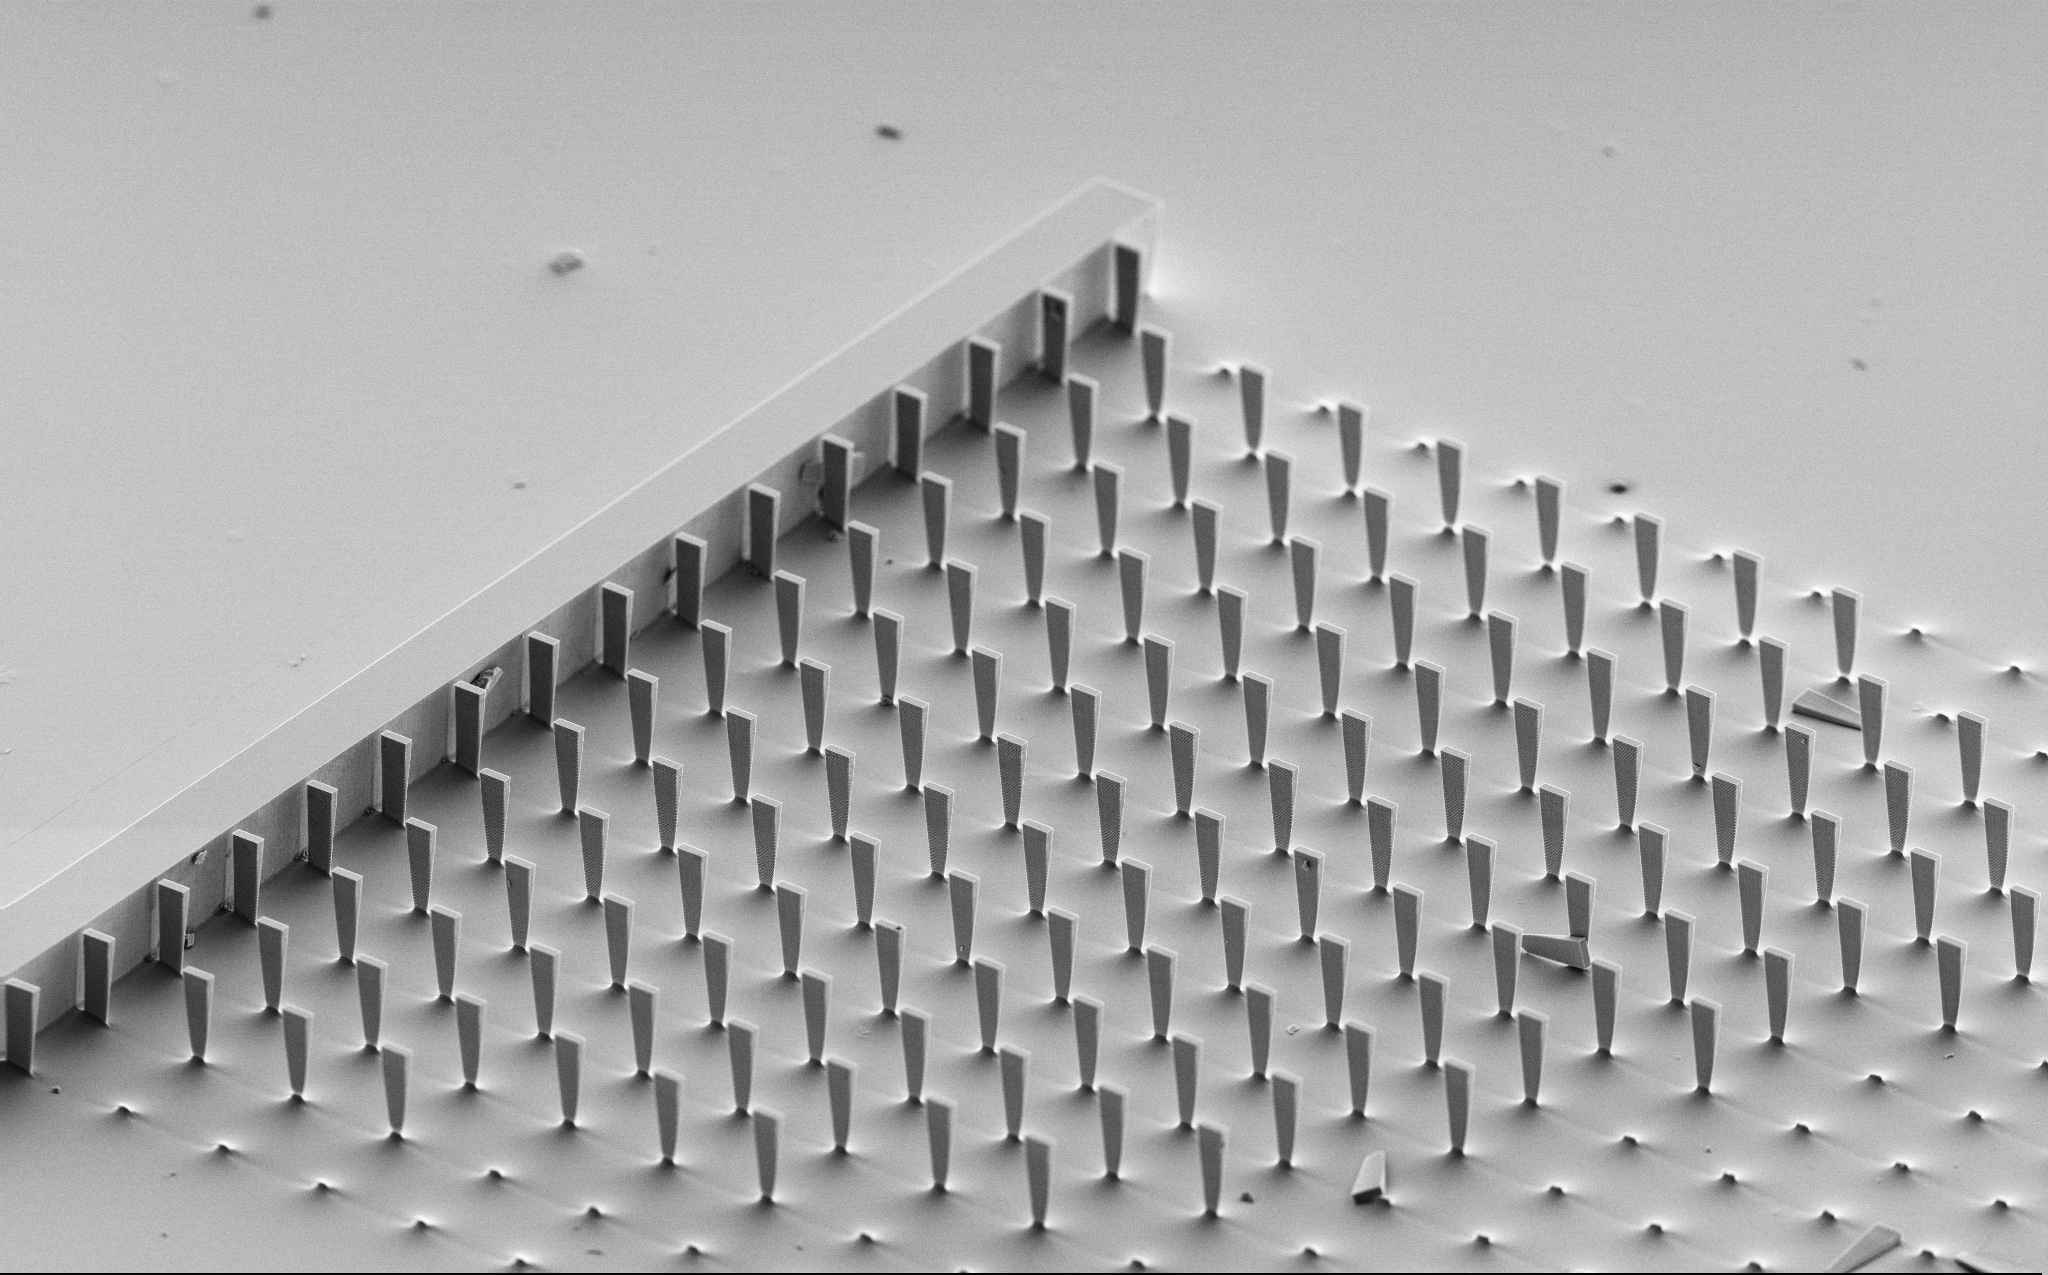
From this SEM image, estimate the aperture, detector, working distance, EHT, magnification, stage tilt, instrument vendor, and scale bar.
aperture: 30 µm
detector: SE2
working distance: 9.7 mm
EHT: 5 kV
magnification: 0.754 K X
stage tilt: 60°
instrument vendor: Zeiss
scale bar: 20000 nm